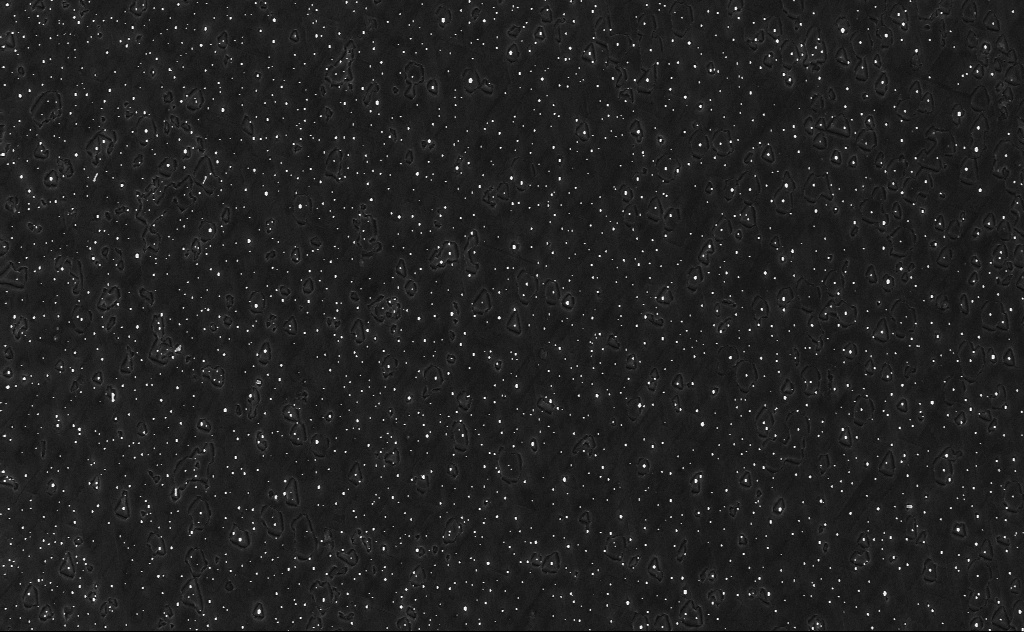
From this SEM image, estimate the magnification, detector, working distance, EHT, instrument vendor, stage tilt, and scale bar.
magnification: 5 K X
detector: InLens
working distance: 6 mm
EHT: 10 kV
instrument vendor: Zeiss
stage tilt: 0°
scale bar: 10000 nm